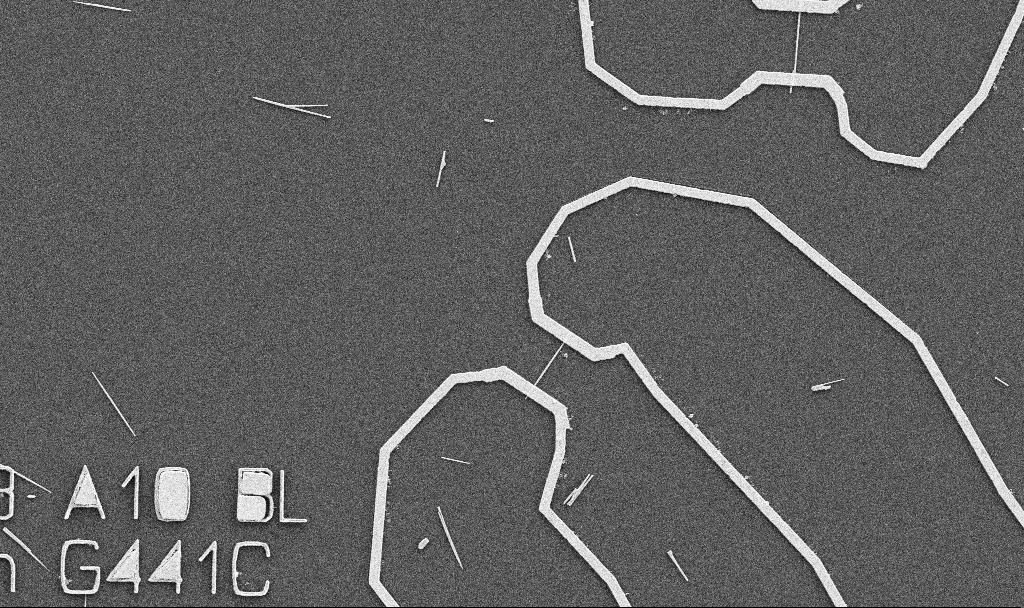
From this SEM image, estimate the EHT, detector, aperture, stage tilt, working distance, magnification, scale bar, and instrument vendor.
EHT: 5 kV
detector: SE2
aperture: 30 µm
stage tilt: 0°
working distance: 10.7 mm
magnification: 5 K X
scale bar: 10000 nm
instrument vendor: Zeiss